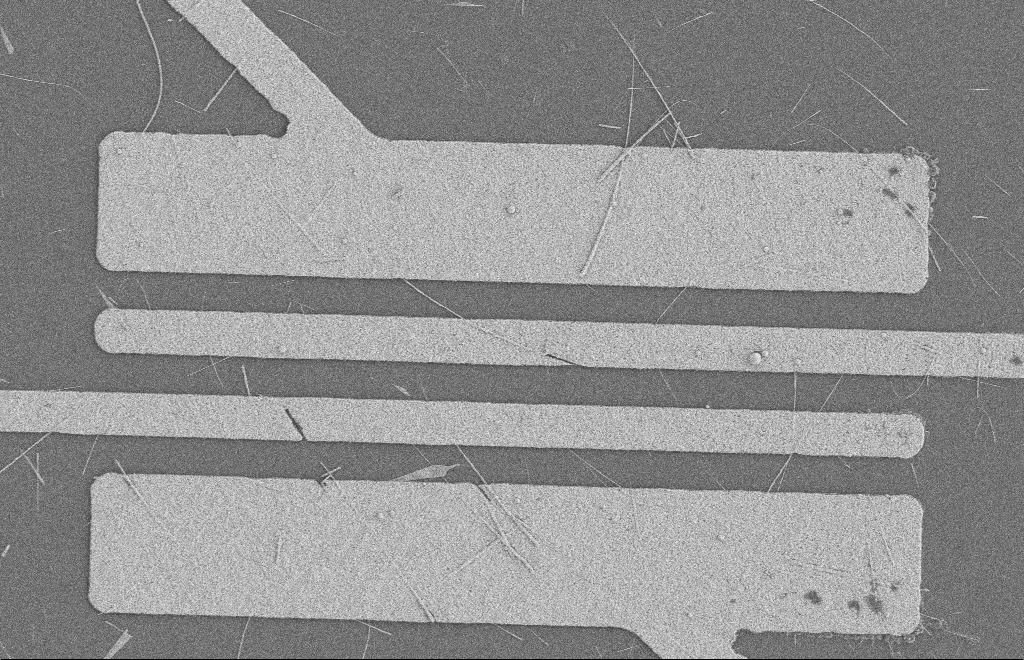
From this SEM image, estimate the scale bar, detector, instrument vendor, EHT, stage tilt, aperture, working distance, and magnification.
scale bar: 2000 nm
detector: SE2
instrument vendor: Zeiss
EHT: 2 kV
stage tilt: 0°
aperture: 20 µm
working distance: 8 mm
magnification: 5.03 K X